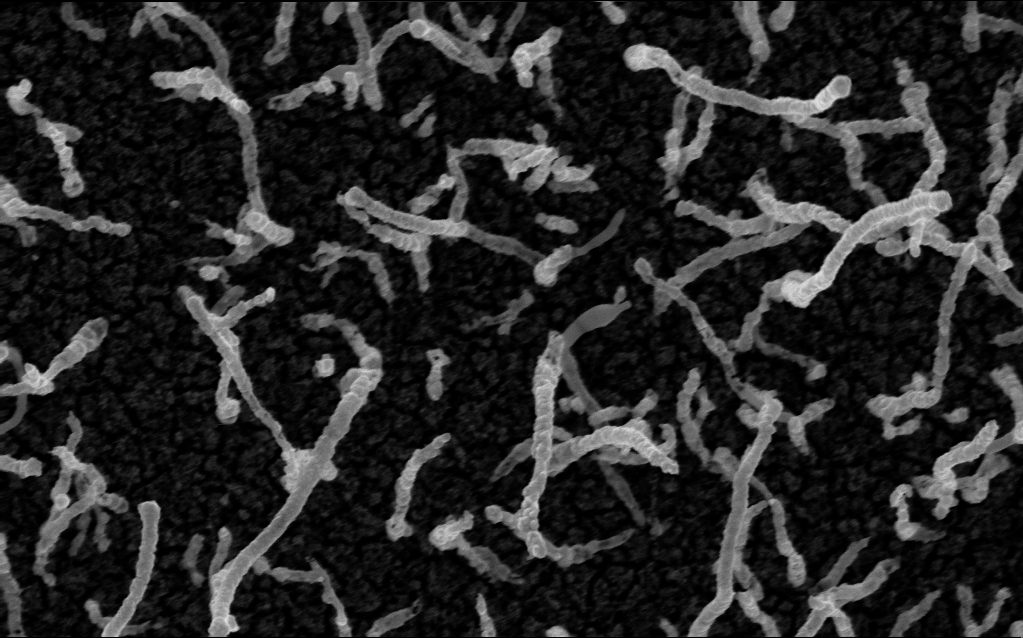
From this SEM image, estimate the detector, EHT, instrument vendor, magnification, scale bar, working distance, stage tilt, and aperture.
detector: InLens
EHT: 5 kV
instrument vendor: Zeiss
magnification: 100 K X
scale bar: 200 nm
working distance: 2.1 mm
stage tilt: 0°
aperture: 30 µm